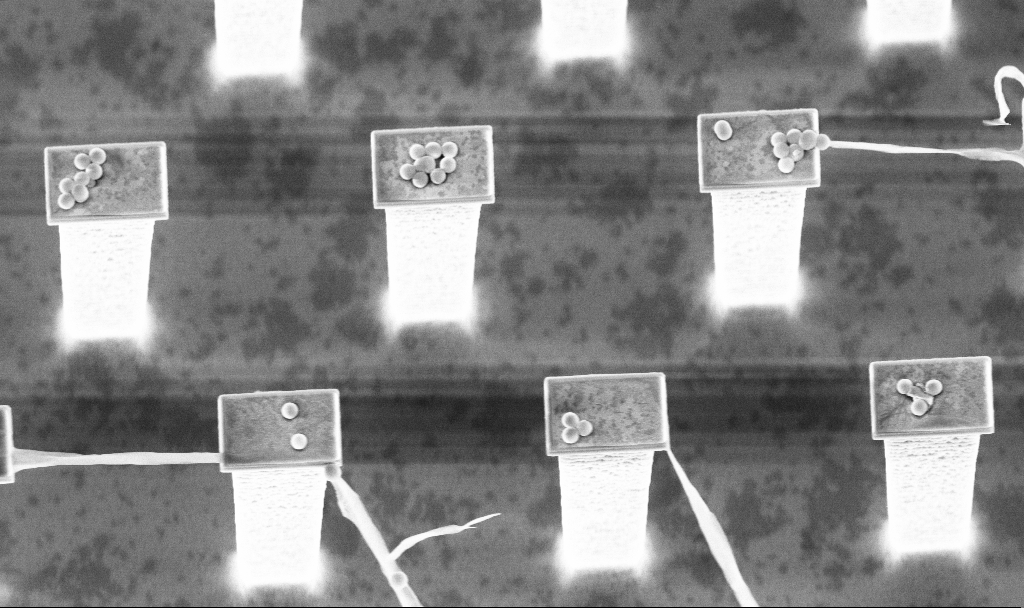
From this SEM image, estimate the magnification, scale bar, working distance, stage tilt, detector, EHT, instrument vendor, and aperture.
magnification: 9.94 K X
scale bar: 2000 nm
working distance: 3.2 mm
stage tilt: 20°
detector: InLens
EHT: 5 kV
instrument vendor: Zeiss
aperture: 30 µm